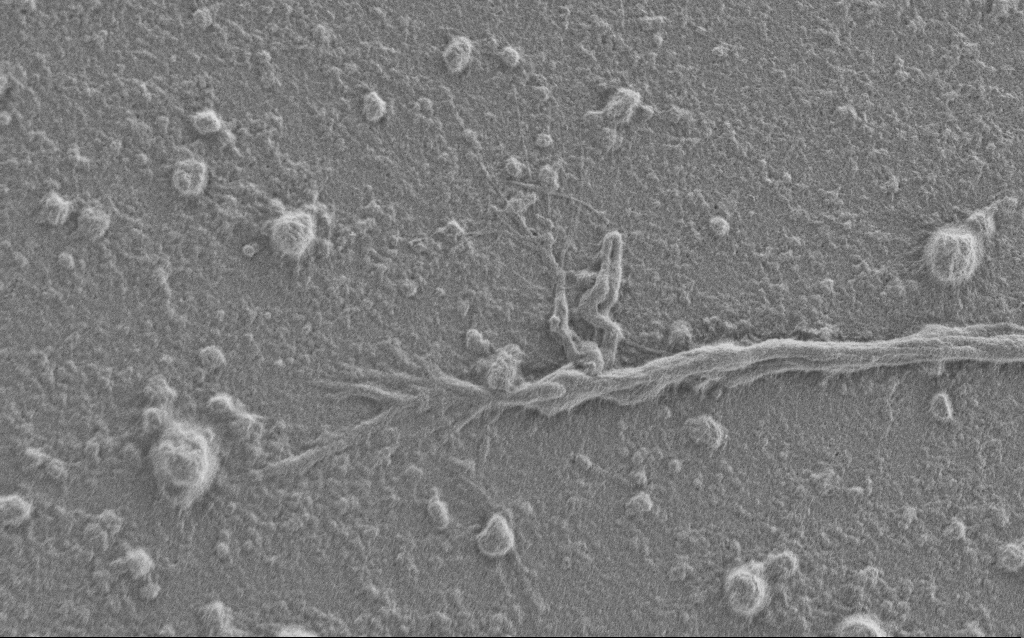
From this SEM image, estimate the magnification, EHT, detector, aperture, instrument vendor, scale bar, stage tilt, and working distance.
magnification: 6.5 K X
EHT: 1 kV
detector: SE2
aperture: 30 µm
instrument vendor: Zeiss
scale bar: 10000 nm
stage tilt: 0°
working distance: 6 mm